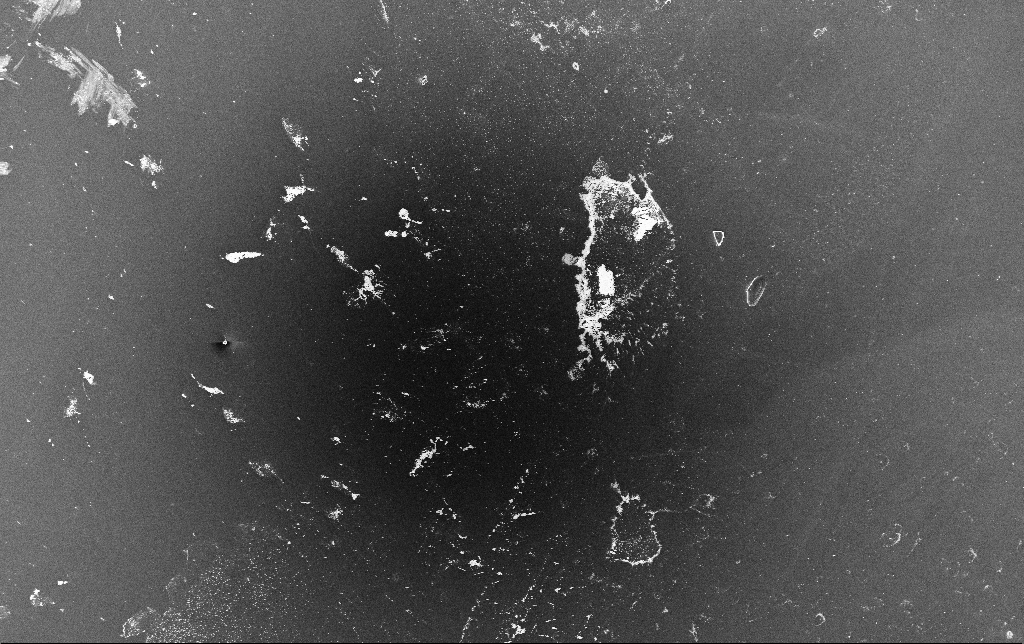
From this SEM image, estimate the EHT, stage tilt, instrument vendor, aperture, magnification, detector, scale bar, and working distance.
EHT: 10 kV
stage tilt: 0°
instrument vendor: Zeiss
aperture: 30 µm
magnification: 0.385 K X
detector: InLens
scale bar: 100000 nm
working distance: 3.4 mm